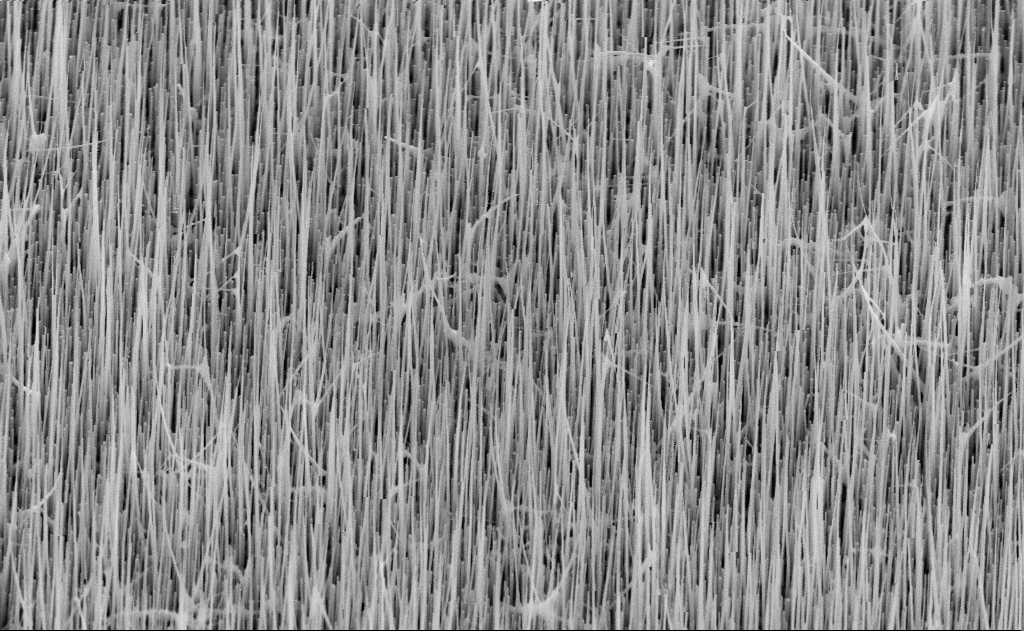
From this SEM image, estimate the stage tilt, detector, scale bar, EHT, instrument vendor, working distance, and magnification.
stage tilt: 45°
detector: SE2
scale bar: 1000 nm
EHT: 10 kV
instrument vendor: Zeiss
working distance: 16 mm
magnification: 20 K X